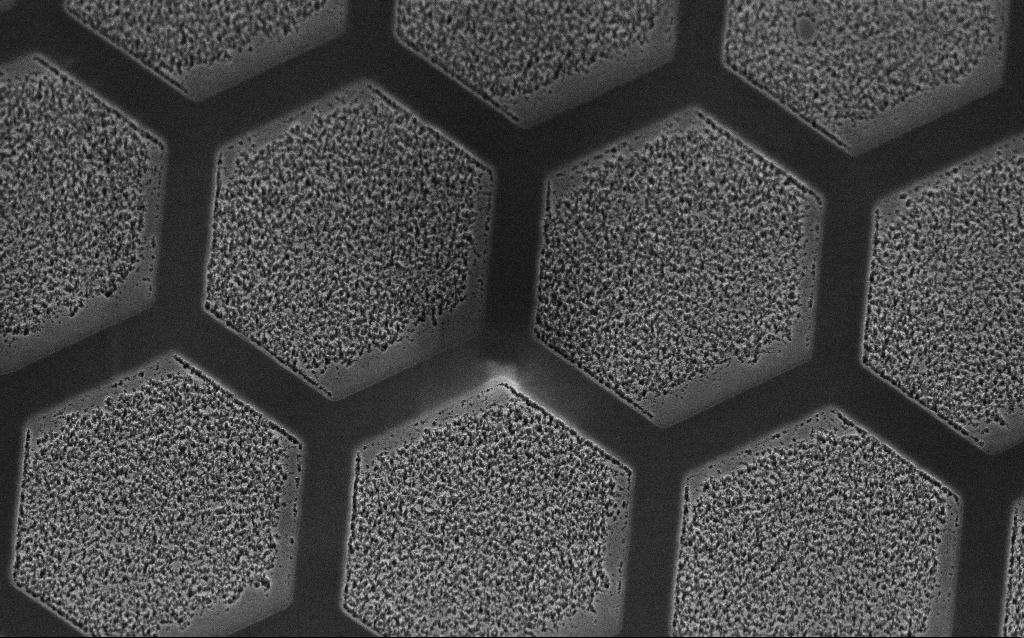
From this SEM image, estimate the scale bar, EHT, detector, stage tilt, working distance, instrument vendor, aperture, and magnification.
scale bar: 1000 nm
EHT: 3 kV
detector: InLens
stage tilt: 45°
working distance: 8 mm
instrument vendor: Zeiss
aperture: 30 µm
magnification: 19.5 K X